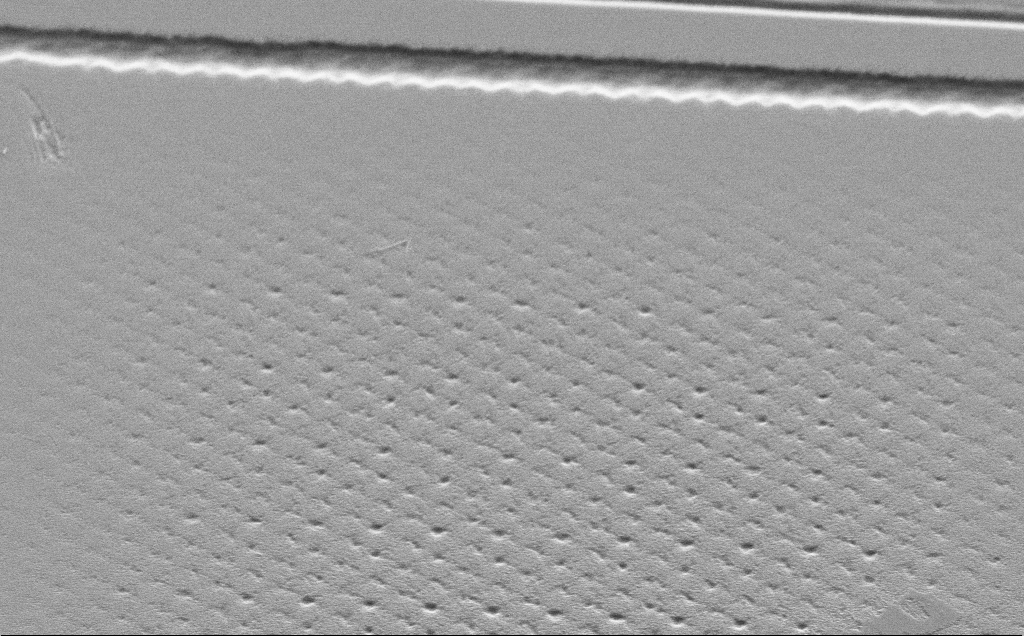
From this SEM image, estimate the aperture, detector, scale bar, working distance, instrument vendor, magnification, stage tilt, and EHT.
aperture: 30 µm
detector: SE2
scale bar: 10000 nm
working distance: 10 mm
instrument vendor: Zeiss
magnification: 5.67 K X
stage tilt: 45°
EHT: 5 kV